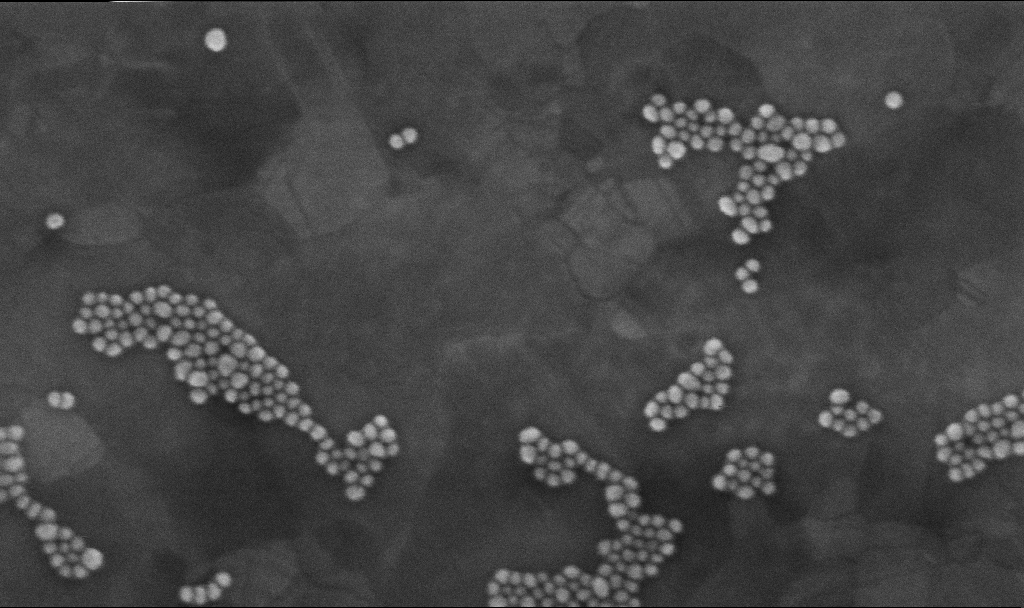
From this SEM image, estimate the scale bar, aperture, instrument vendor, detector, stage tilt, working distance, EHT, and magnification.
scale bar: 200 nm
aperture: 30 µm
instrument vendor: Zeiss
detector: InLens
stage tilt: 0°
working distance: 3.2 mm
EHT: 10 kV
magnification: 300 K X